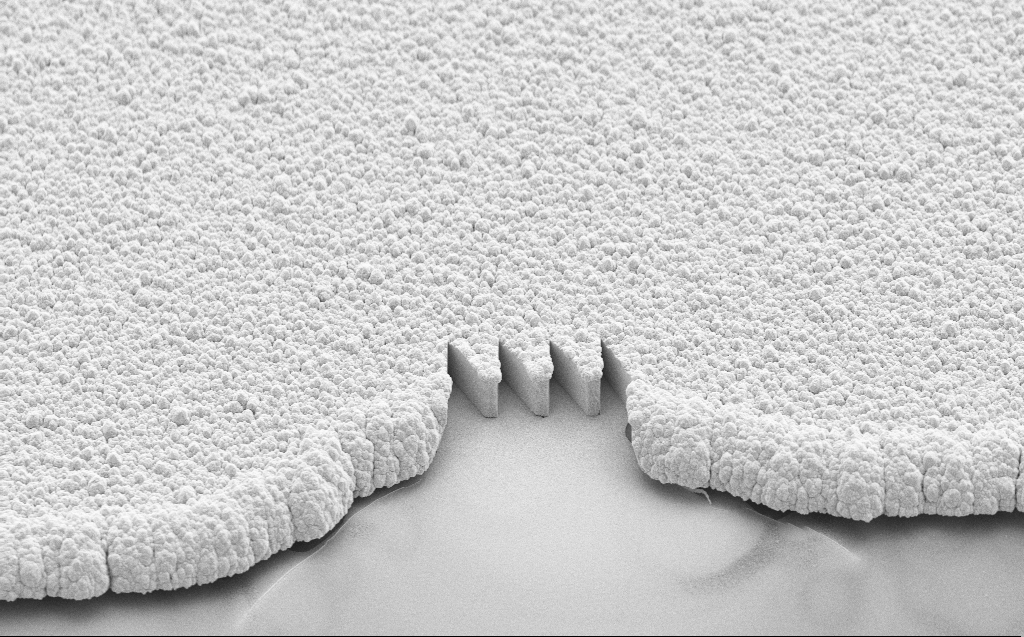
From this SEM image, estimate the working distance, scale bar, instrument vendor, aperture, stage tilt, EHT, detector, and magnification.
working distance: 7 mm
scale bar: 10000 nm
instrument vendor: Zeiss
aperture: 30 µm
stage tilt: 44.5°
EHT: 10 kV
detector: SE2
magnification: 1.63 K X